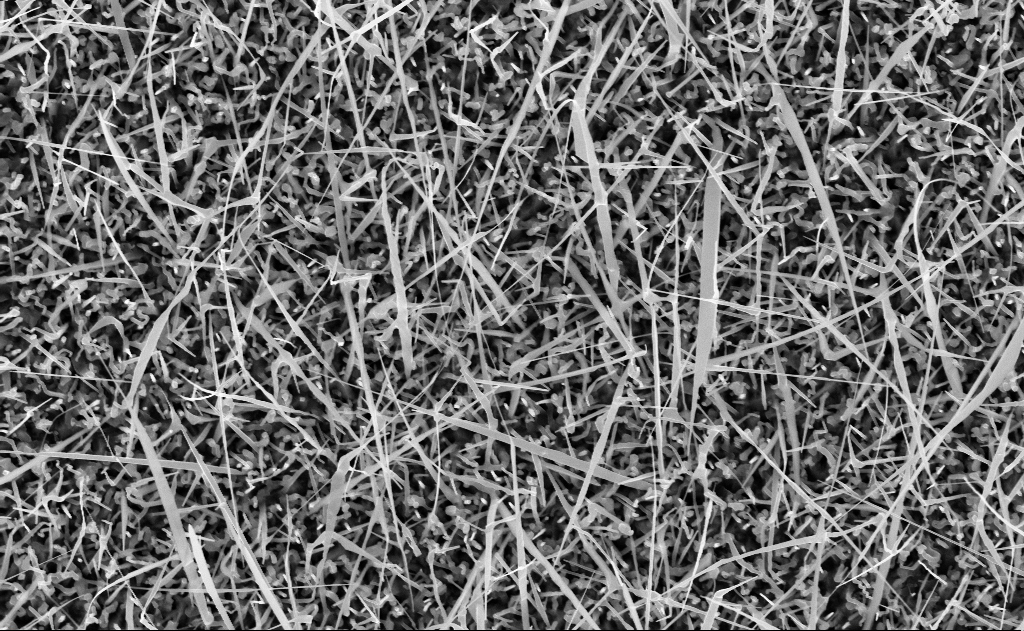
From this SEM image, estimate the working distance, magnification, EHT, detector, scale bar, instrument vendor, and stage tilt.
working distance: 10 mm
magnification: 20 K X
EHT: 10 kV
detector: InLens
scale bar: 2000 nm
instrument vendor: Zeiss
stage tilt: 0°